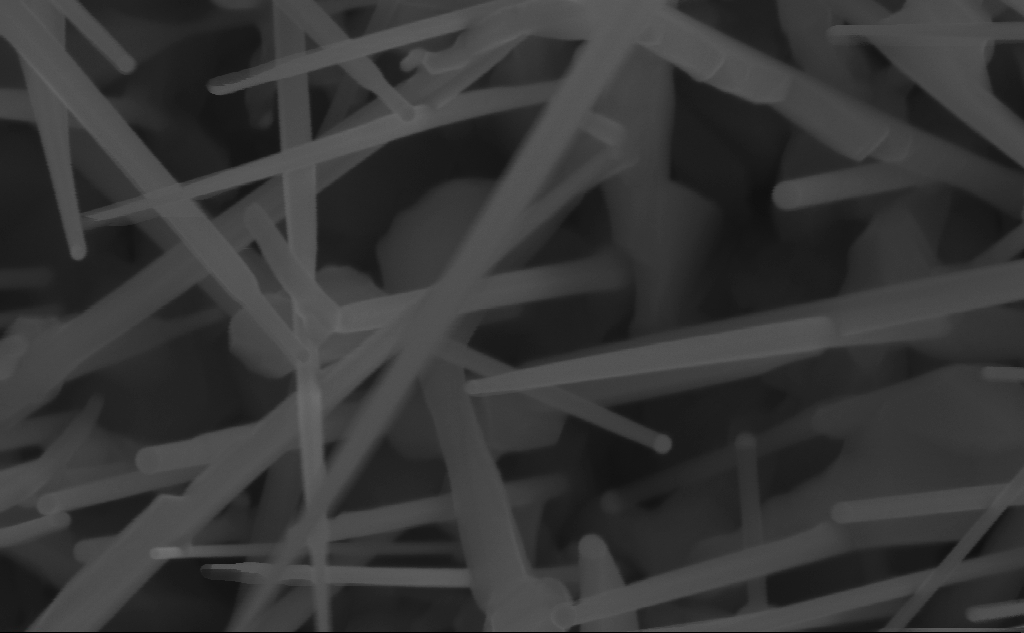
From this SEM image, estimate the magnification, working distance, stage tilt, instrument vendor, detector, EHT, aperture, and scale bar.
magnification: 196.56 K X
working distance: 7 mm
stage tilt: -0°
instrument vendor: Zeiss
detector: InLens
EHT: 10 kV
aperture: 30 µm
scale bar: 200 nm